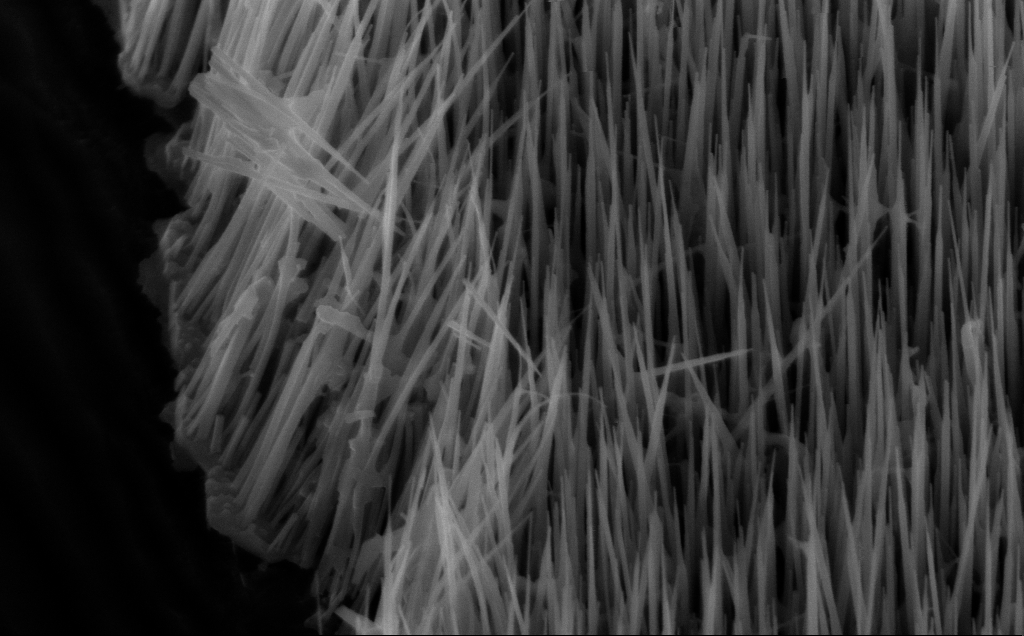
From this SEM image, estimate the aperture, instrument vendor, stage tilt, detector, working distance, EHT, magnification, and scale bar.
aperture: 30 µm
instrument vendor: Zeiss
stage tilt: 45°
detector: InLens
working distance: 8 mm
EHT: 10 kV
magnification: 76.05 K X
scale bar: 200 nm